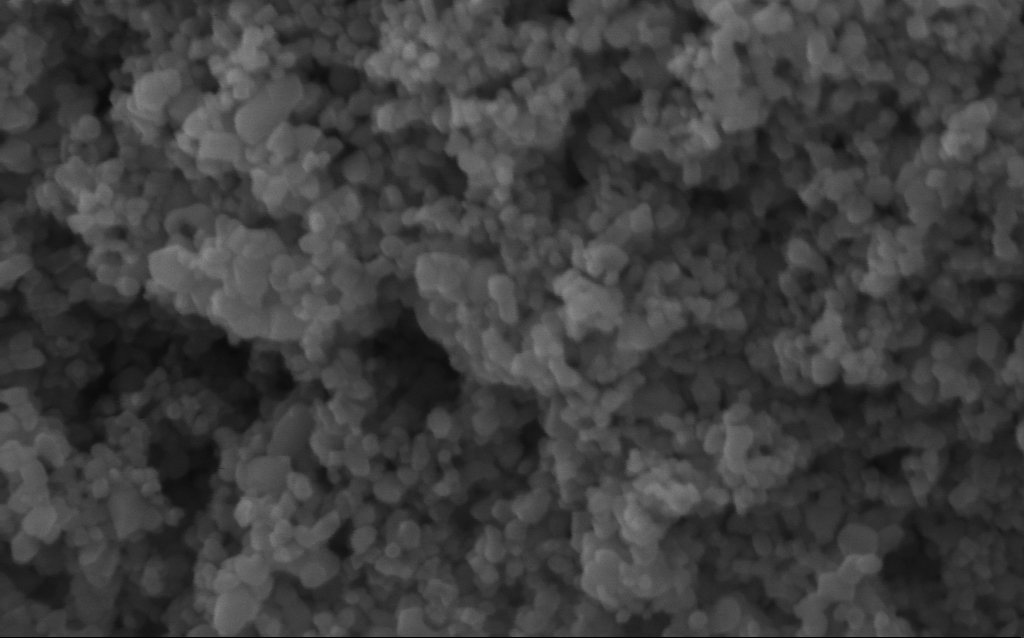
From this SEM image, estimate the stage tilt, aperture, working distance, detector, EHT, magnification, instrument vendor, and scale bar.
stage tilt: -0°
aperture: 30 µm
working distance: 4.2 mm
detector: InLens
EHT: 5 kV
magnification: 282.07 K X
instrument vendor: Zeiss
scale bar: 200 nm